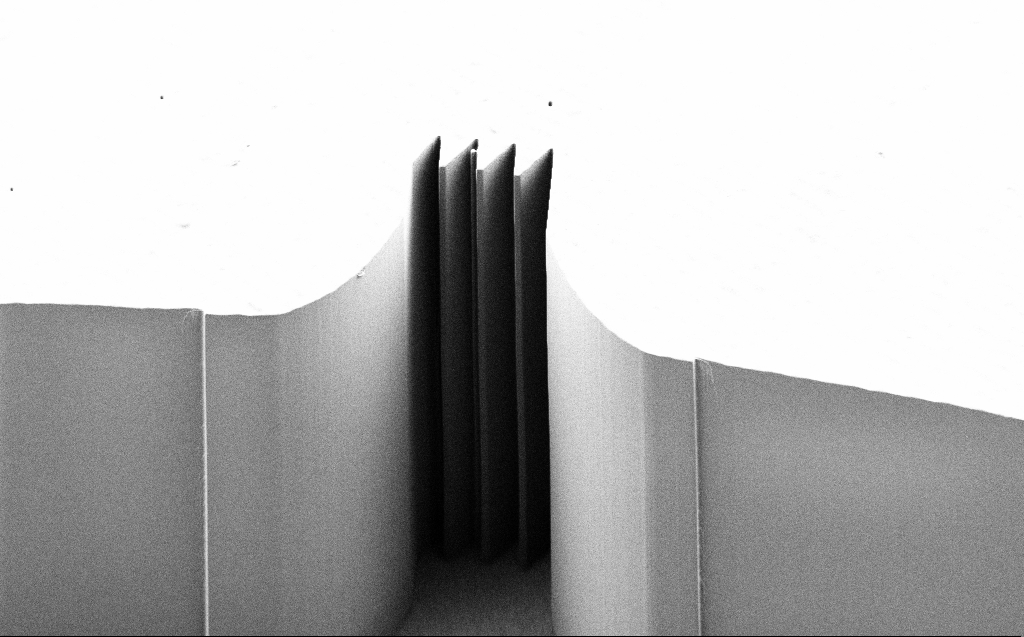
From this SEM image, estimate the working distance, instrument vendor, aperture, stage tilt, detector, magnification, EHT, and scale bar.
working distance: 7 mm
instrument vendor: Zeiss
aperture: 30 µm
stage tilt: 44.9°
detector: SE2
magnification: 1.21 K X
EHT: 1 kV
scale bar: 10000 nm